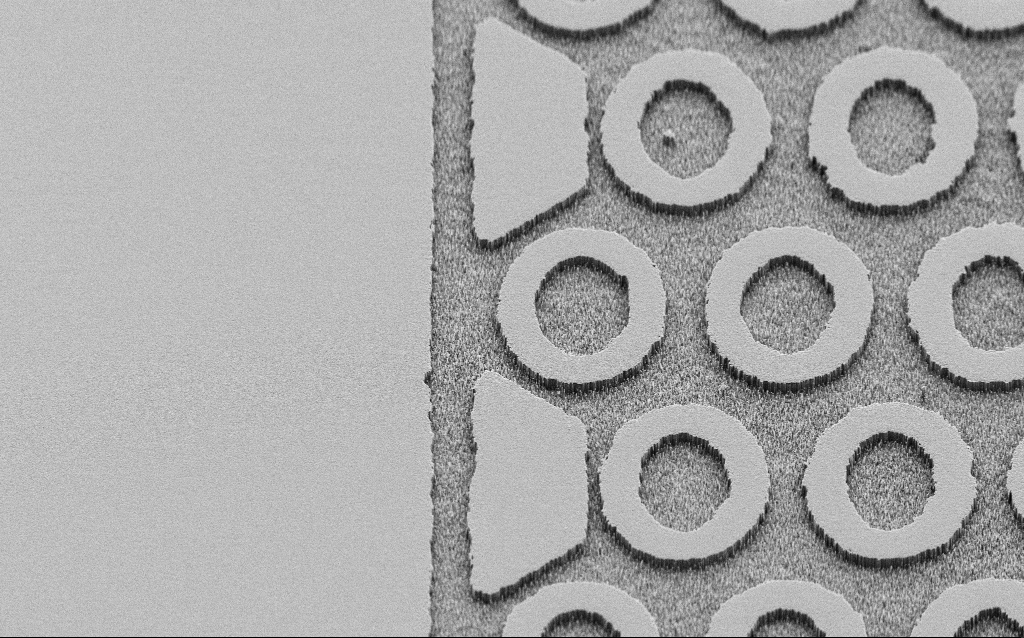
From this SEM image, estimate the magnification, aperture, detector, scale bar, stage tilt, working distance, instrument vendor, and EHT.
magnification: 0.949 K X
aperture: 30 µm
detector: SE2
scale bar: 20000 nm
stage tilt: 45°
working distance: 7 mm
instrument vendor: Zeiss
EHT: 2 kV